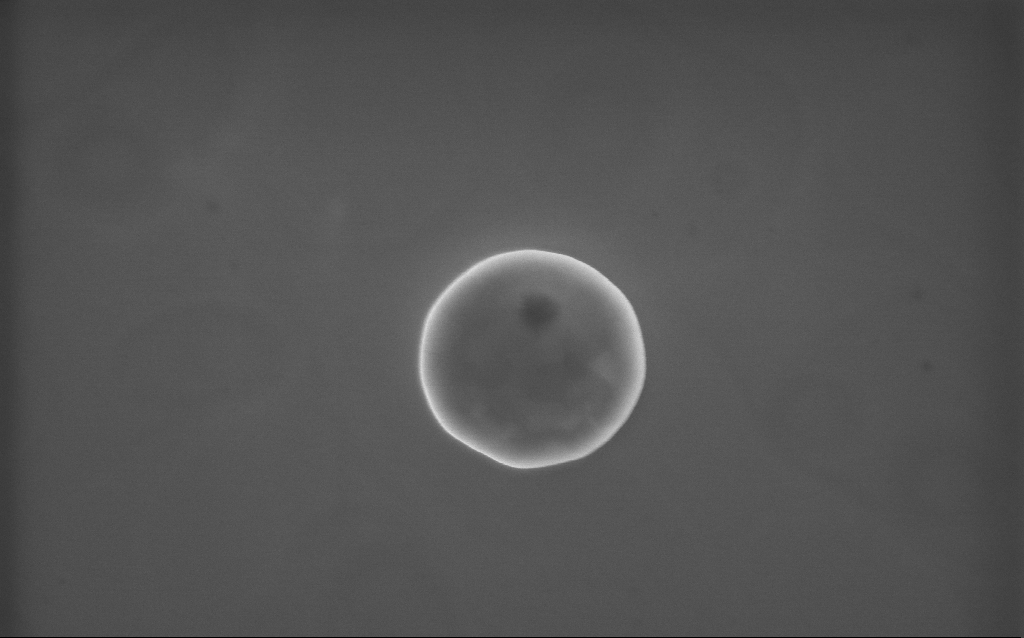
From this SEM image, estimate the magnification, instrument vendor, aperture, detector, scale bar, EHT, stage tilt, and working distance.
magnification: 65 K X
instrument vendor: Zeiss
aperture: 30 µm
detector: InLens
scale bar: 1000 nm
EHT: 10 kV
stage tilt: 0°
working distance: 2 mm